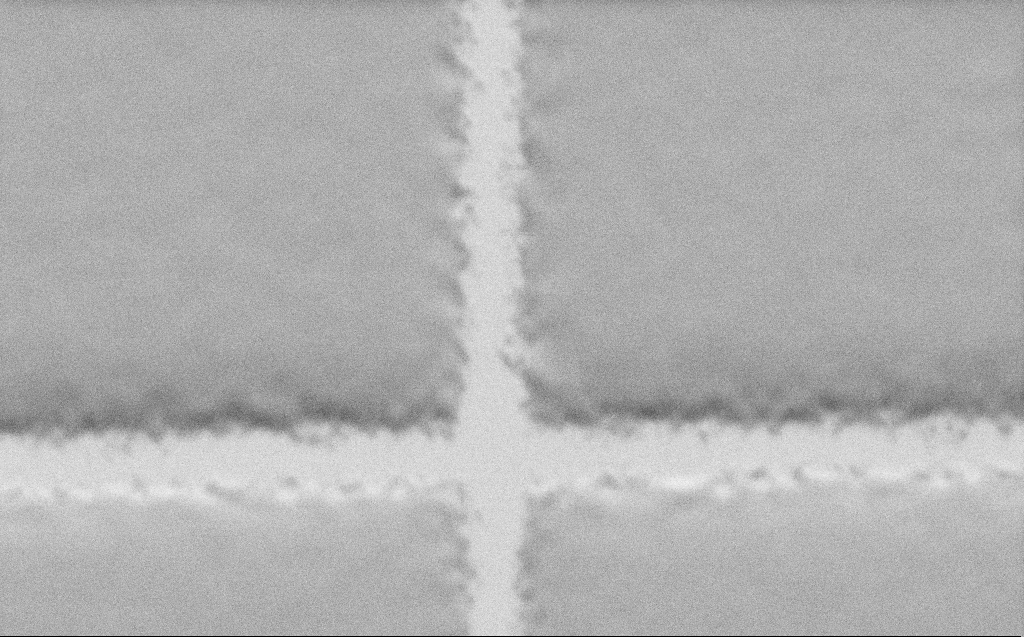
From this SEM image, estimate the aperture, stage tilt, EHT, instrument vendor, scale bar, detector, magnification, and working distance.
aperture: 30 µm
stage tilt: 45°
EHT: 1 kV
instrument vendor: Zeiss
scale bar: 2000 nm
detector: SE2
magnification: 28.56 K X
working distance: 5 mm